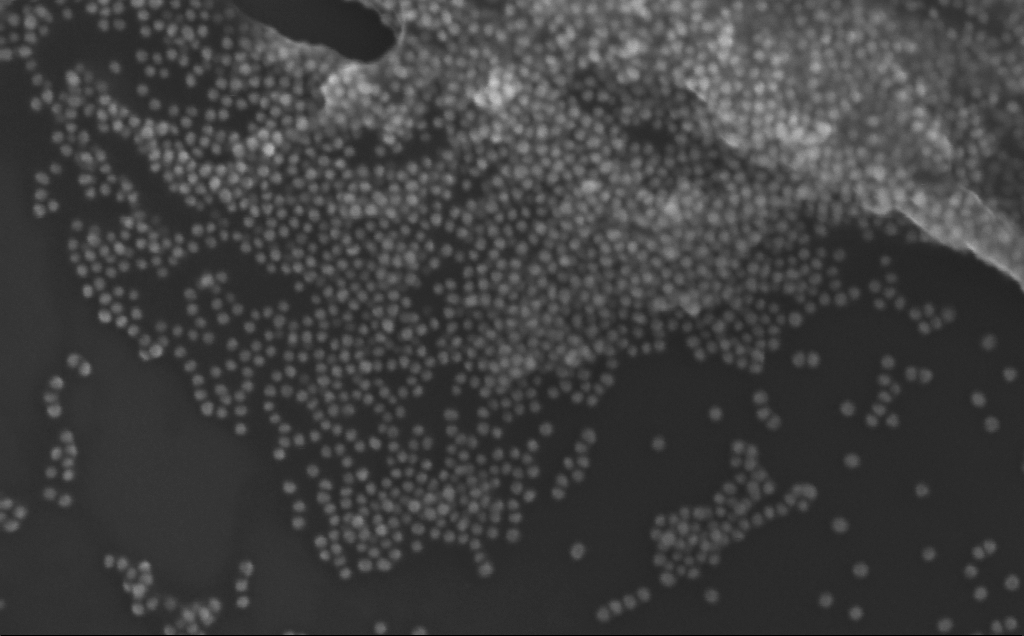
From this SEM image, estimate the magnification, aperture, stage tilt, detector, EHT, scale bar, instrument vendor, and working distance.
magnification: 283.86 K X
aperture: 30 µm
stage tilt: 0°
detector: InLens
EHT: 10 kV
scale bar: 200 nm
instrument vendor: Zeiss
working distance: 3 mm